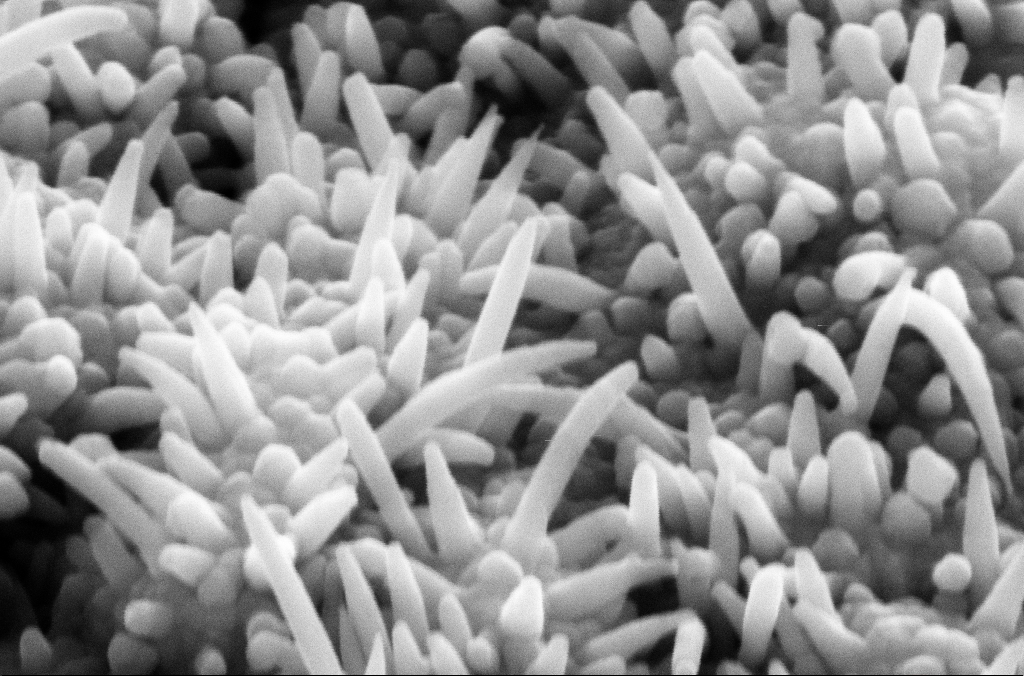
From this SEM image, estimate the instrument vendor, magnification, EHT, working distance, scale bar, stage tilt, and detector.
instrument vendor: Zeiss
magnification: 208.81 K X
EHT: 10 kV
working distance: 6.6 mm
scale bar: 200 nm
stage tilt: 45°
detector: SE2